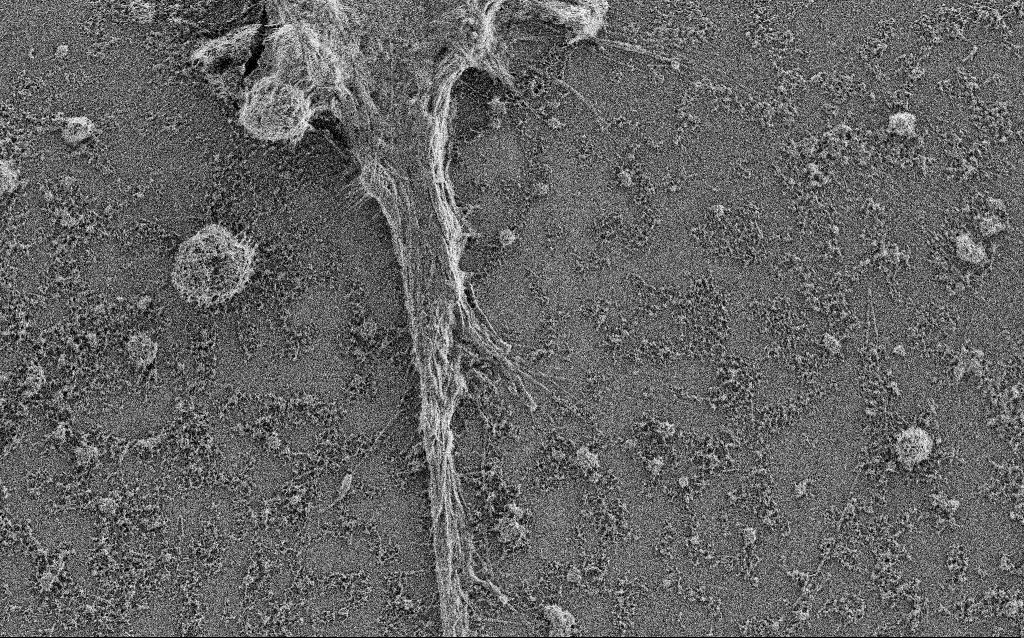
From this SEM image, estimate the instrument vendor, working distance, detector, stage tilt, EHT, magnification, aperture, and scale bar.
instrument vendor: Zeiss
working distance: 4 mm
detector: SE2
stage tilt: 0°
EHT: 0.9 kV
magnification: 5 K X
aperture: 30 µm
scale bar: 10000 nm